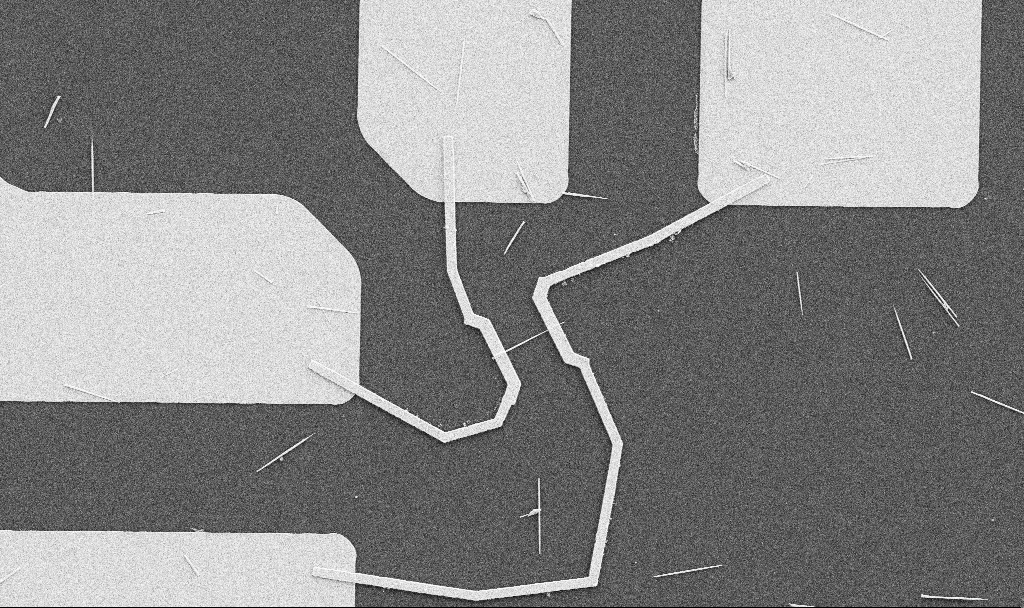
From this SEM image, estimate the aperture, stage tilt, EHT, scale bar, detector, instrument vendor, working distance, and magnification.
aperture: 30 µm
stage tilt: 0°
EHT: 5 kV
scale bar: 10000 nm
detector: SE2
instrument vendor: Zeiss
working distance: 10.7 mm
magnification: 5 K X